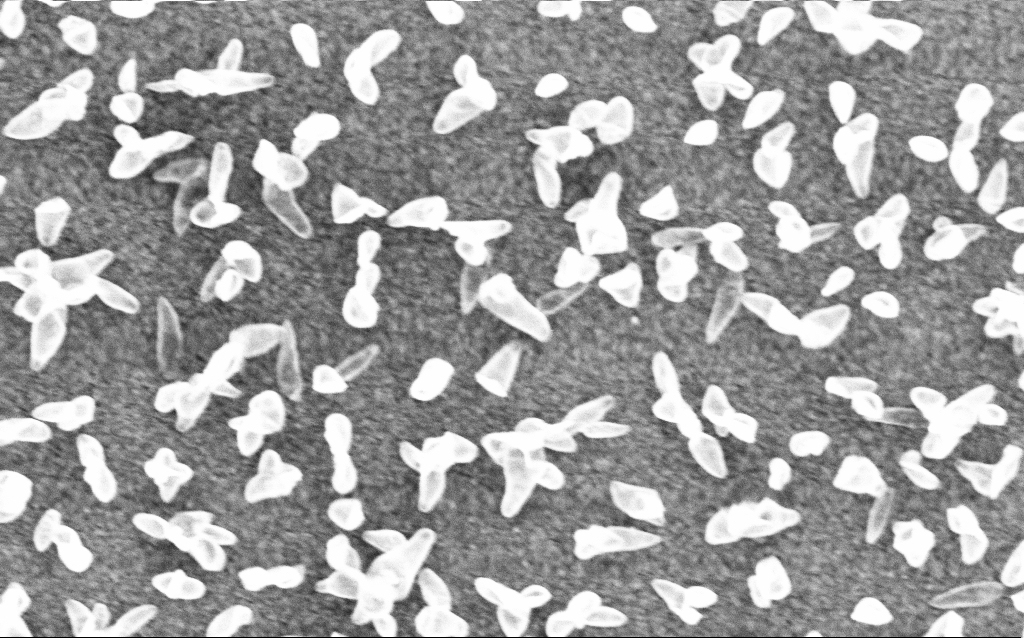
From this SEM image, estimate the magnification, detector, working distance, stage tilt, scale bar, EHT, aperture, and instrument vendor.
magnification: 77.69 K X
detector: InLens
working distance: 6 mm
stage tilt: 0°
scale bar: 200 nm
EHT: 5 kV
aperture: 30 µm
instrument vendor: Zeiss